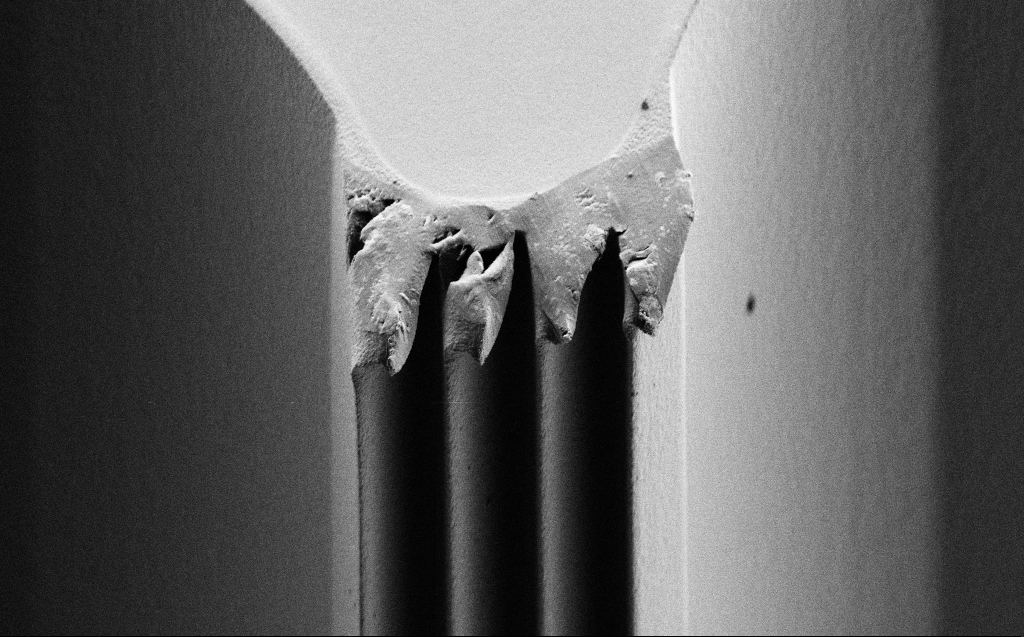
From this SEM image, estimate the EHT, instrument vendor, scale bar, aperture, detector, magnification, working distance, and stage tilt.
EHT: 1 kV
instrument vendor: Zeiss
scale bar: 20000 nm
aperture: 30 µm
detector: SE2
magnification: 2.94 K X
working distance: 5 mm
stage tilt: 44.6°